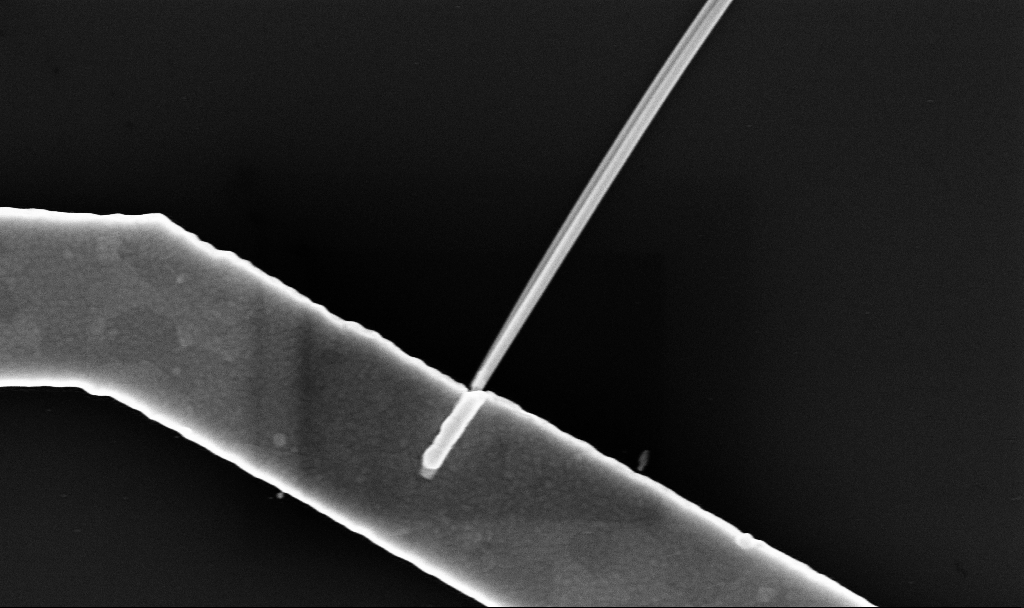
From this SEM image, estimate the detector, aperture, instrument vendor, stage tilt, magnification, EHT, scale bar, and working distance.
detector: InLens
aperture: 30 µm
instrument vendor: Zeiss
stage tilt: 0°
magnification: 64.72 K X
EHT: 10 kV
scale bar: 1000 nm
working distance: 6.7 mm